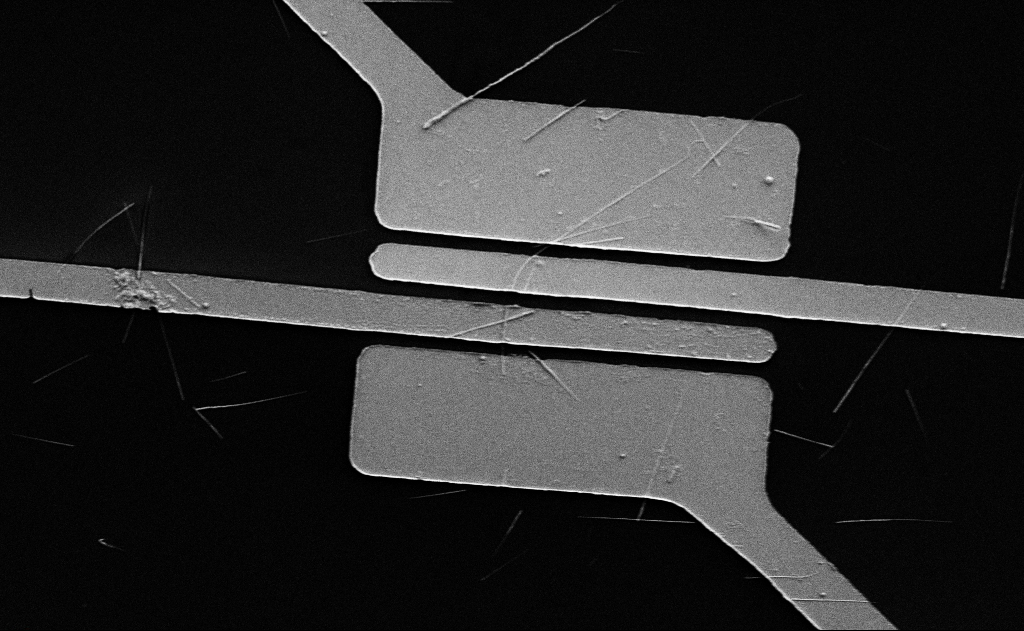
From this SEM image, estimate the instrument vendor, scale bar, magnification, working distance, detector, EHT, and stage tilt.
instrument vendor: Zeiss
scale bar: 10000 nm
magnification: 5 K X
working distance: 15 mm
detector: SE2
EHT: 5 kV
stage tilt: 0°